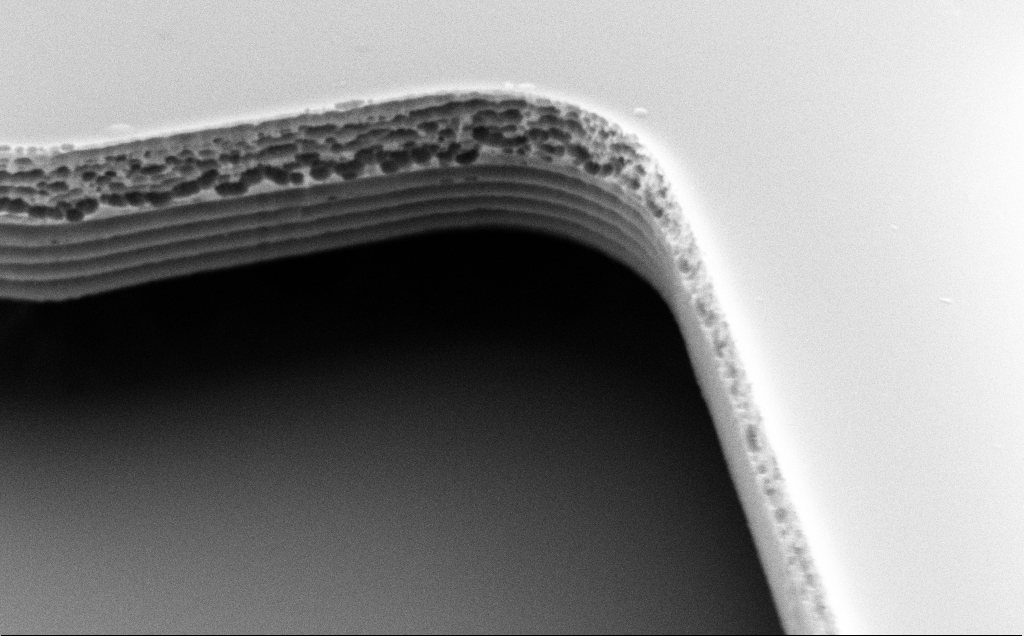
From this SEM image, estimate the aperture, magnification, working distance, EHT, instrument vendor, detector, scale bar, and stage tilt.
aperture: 30 µm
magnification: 33.82 K X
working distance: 10 mm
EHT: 5 kV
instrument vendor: Zeiss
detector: SE2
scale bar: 1000 nm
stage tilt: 50°